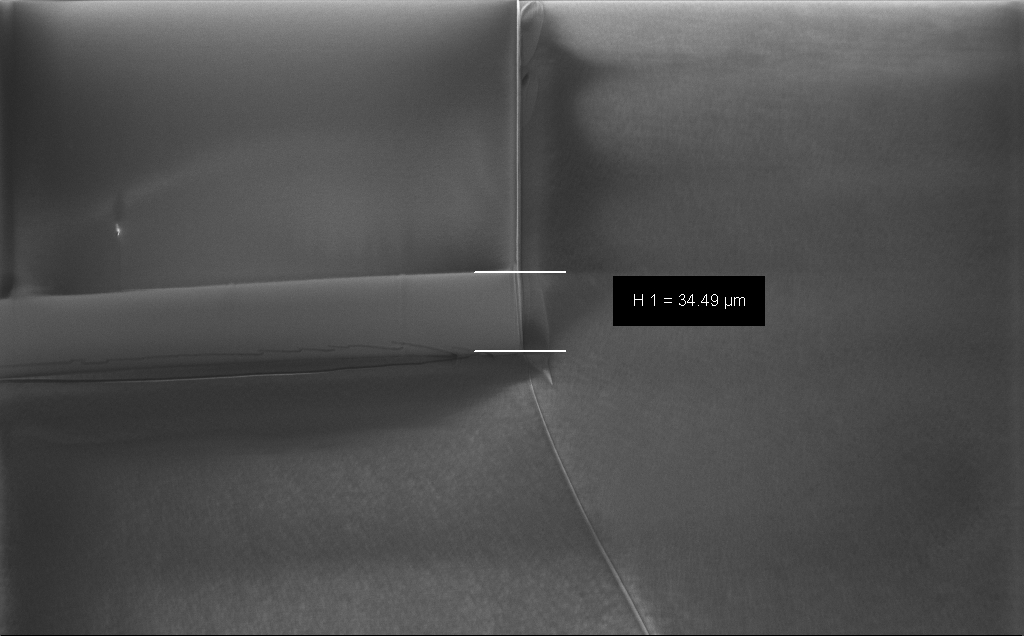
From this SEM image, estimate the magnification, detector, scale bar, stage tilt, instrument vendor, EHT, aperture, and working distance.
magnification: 0.841 K X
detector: InLens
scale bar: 20000 nm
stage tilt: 30°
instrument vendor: Zeiss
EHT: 1.2 kV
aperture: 30 µm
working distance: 8 mm